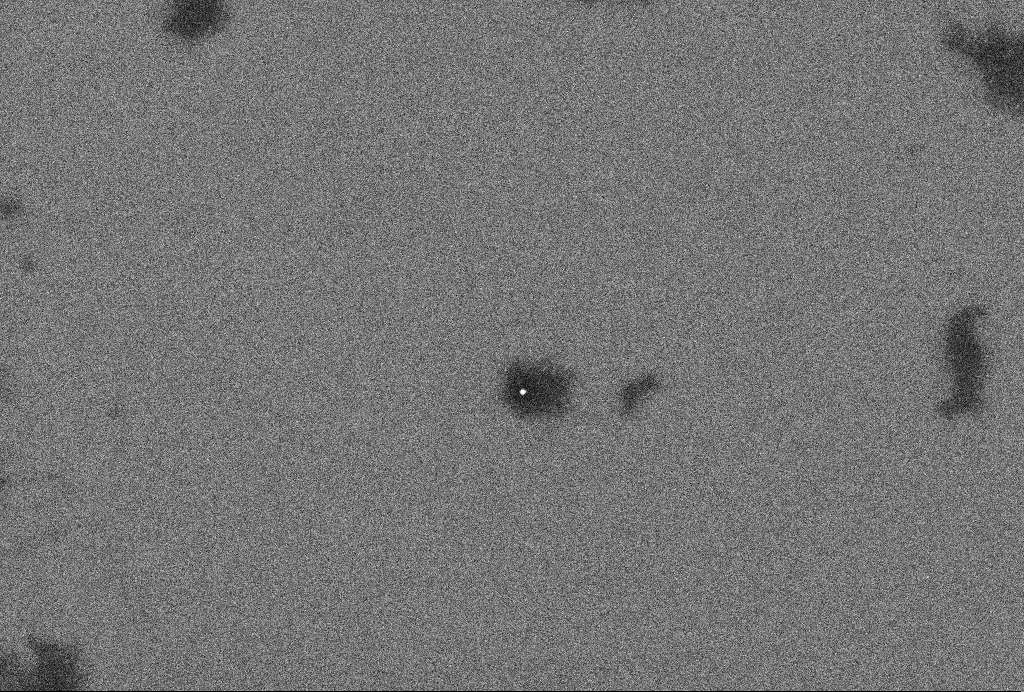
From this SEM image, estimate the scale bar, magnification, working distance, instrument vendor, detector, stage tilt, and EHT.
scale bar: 200 nm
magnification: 82.67 K X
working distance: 3.3 mm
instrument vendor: Zeiss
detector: InLens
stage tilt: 0°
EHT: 2 kV